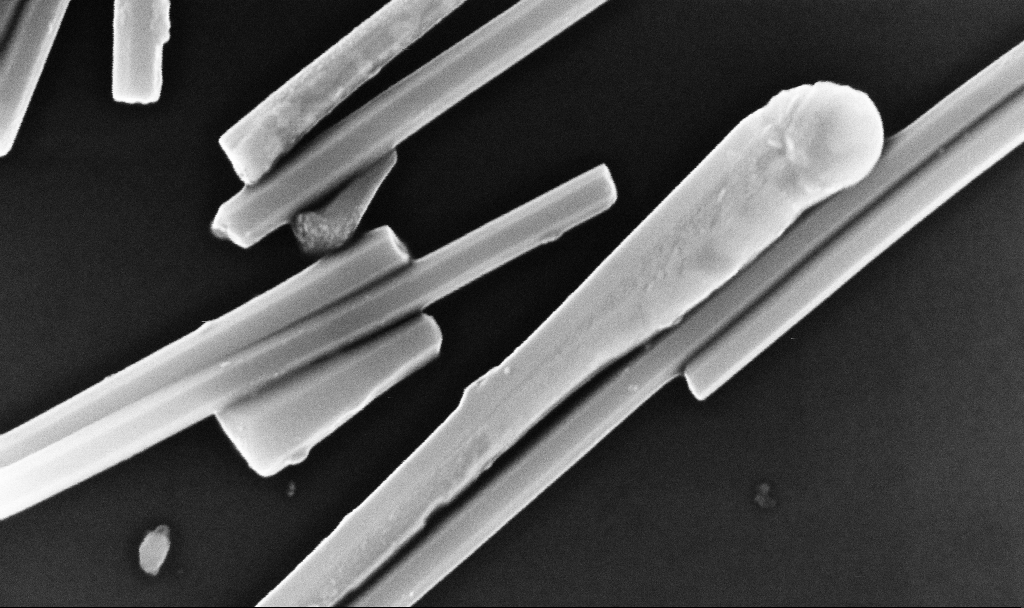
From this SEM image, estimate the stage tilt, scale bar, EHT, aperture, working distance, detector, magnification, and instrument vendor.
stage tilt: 0°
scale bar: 200 nm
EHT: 10 kV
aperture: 30 µm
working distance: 6.7 mm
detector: InLens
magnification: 159.66 K X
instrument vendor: Zeiss